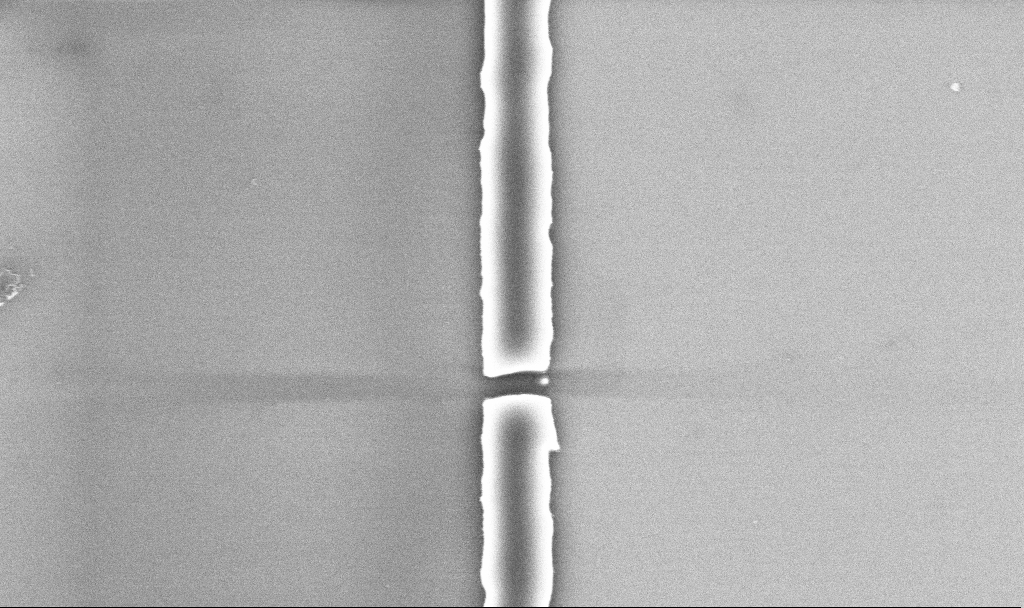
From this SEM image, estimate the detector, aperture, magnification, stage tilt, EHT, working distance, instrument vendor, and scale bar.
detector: InLens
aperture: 30 µm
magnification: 45.07 K X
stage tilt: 0°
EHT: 5 kV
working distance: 5.2 mm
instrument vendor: Zeiss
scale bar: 1000 nm